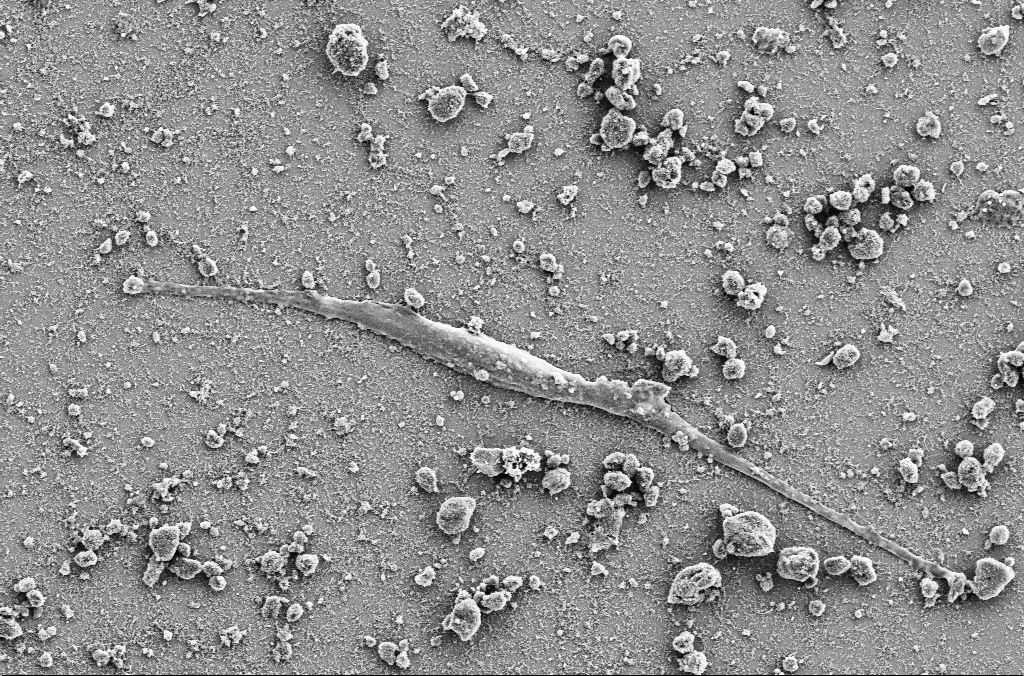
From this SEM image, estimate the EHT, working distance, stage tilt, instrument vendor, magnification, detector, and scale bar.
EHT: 5 kV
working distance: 4 mm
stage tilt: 0°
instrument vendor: Zeiss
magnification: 3 K X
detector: SE2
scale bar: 10000 nm